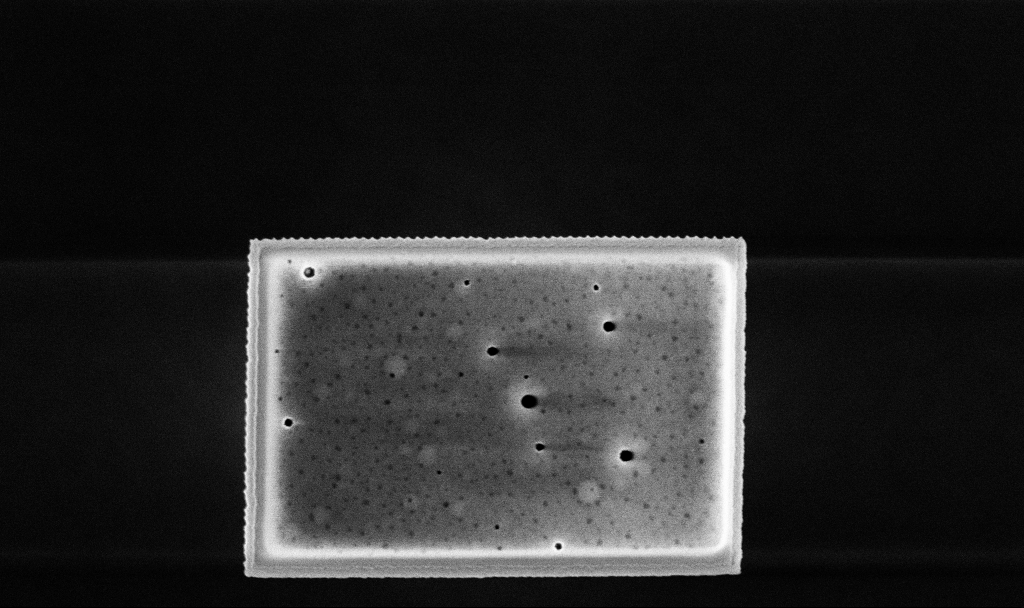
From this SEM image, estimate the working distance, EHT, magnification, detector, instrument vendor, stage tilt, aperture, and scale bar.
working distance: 3.3 mm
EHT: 5 kV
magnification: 42.2 K X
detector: InLens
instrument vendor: Zeiss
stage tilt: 0°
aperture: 30 µm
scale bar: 1000 nm